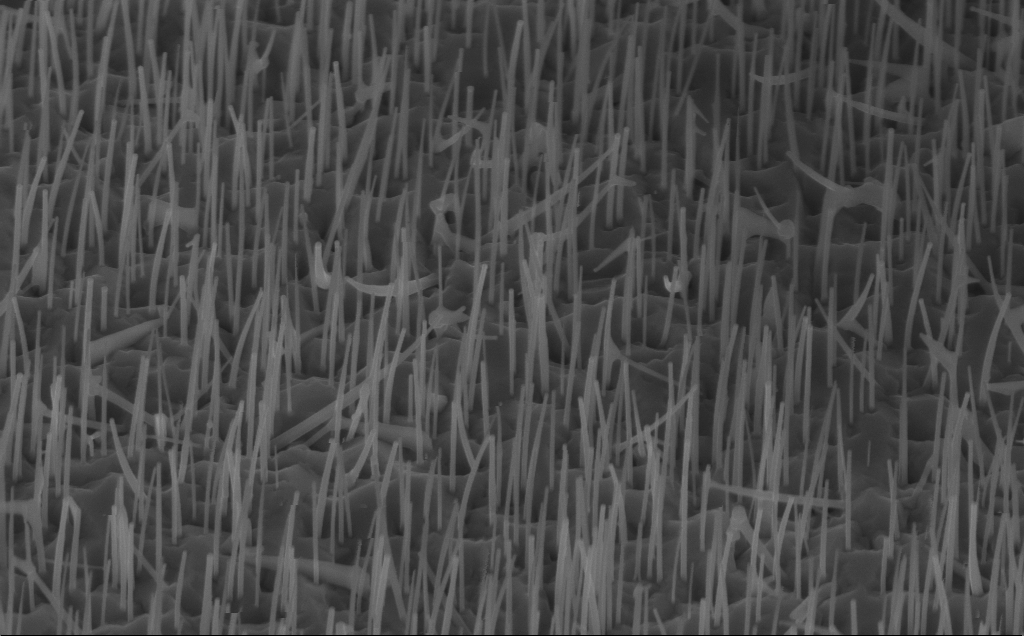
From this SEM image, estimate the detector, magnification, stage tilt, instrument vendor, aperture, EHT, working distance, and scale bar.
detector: InLens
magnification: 40 K X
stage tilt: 45°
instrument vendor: Zeiss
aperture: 30 µm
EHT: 10 kV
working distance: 7 mm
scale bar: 1000 nm